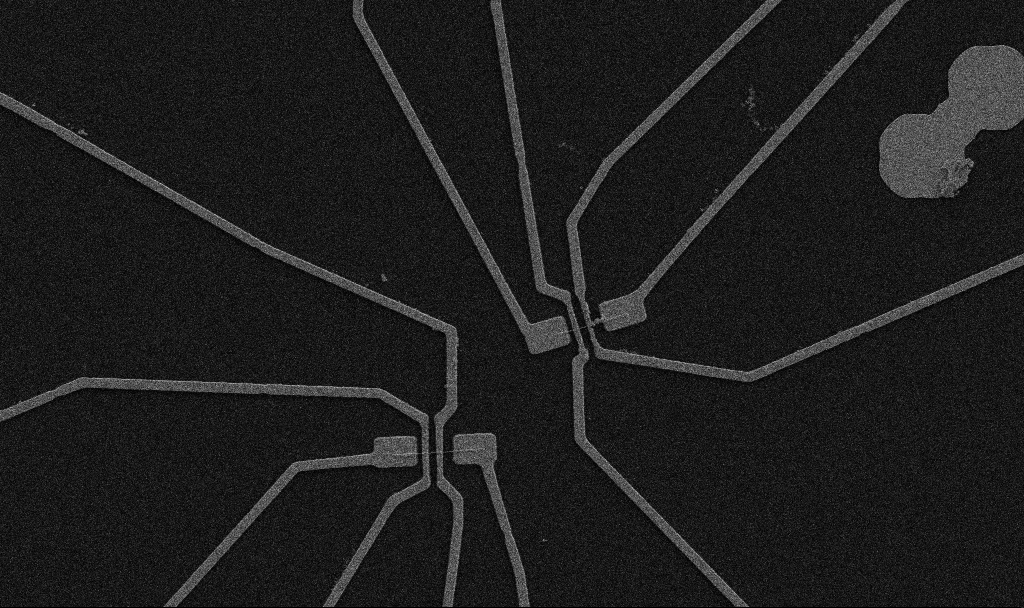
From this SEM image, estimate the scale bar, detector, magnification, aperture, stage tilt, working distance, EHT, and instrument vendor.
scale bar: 10000 nm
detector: SE2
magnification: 5 K X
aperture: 30 µm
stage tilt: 0°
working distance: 10.7 mm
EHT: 5 kV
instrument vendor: Zeiss